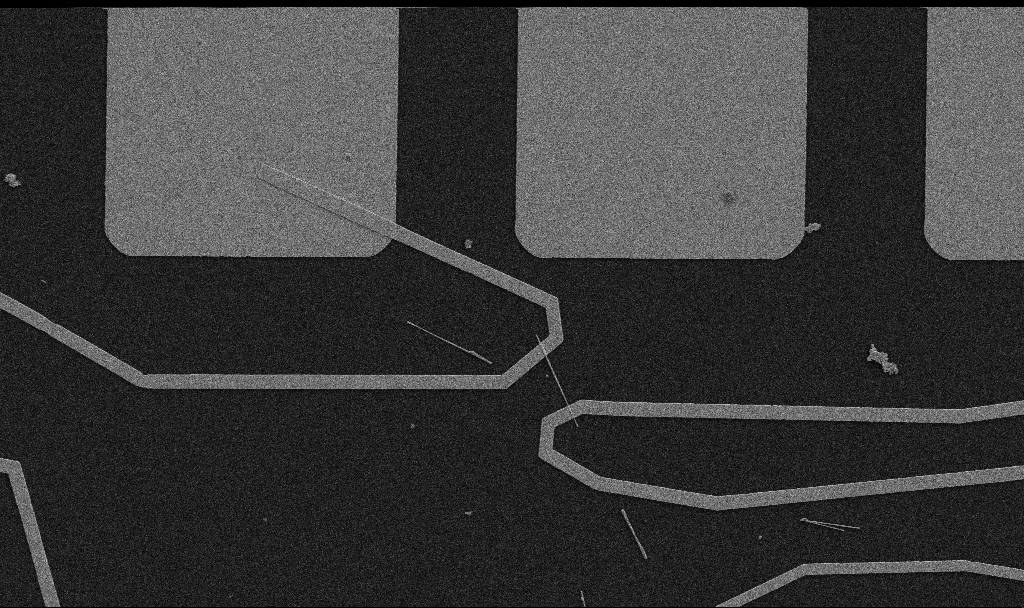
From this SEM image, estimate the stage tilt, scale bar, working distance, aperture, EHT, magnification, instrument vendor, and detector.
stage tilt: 0°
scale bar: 10000 nm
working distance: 10.7 mm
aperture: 30 µm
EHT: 5 kV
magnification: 5 K X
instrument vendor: Zeiss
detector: SE2